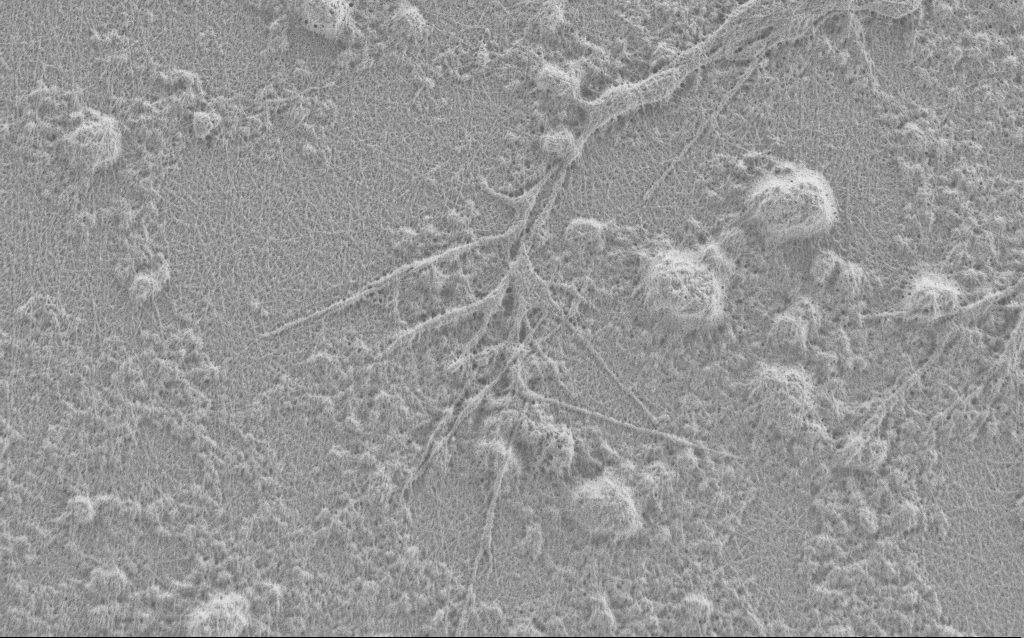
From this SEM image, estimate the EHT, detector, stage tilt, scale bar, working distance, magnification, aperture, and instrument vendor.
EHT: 0.9 kV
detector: SE2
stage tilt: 0°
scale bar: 2000 nm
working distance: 4 mm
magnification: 10 K X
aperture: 30 µm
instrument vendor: Zeiss